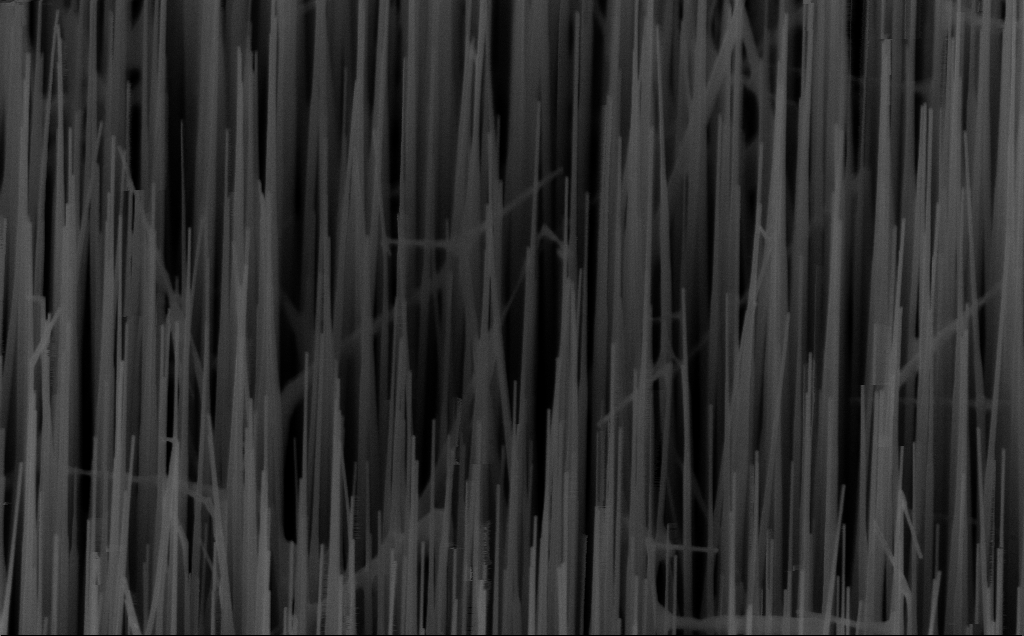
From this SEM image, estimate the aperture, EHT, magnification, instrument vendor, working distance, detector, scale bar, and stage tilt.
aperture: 30 µm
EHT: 10 kV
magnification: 40 K X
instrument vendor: Zeiss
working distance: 6 mm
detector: InLens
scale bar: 1000 nm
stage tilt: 45°